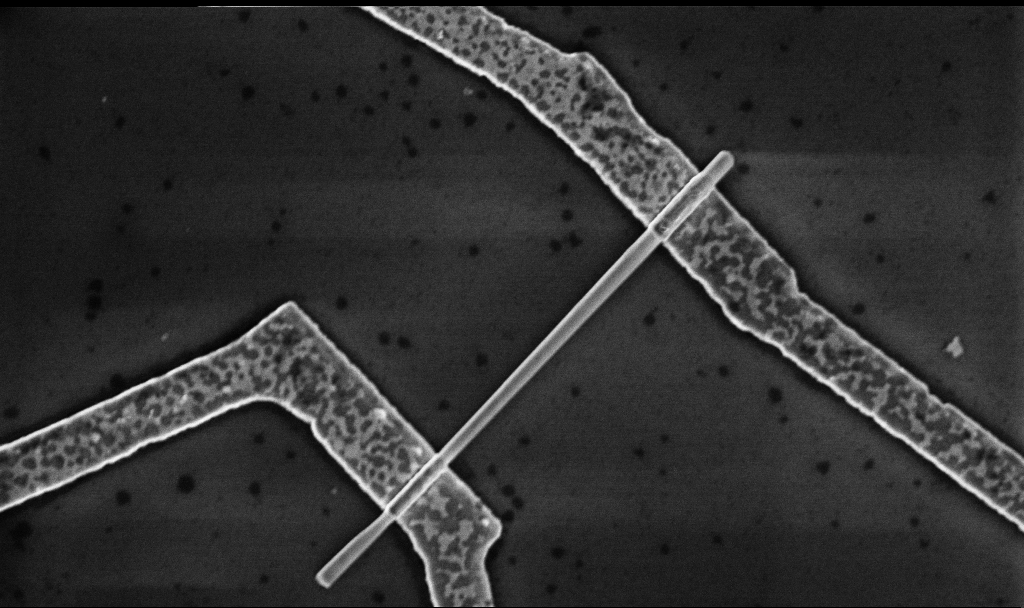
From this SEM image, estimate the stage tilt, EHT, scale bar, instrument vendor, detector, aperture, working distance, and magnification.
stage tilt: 0°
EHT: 5 kV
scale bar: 1000 nm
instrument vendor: Zeiss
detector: InLens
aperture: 30 µm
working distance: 8.7 mm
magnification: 30 K X